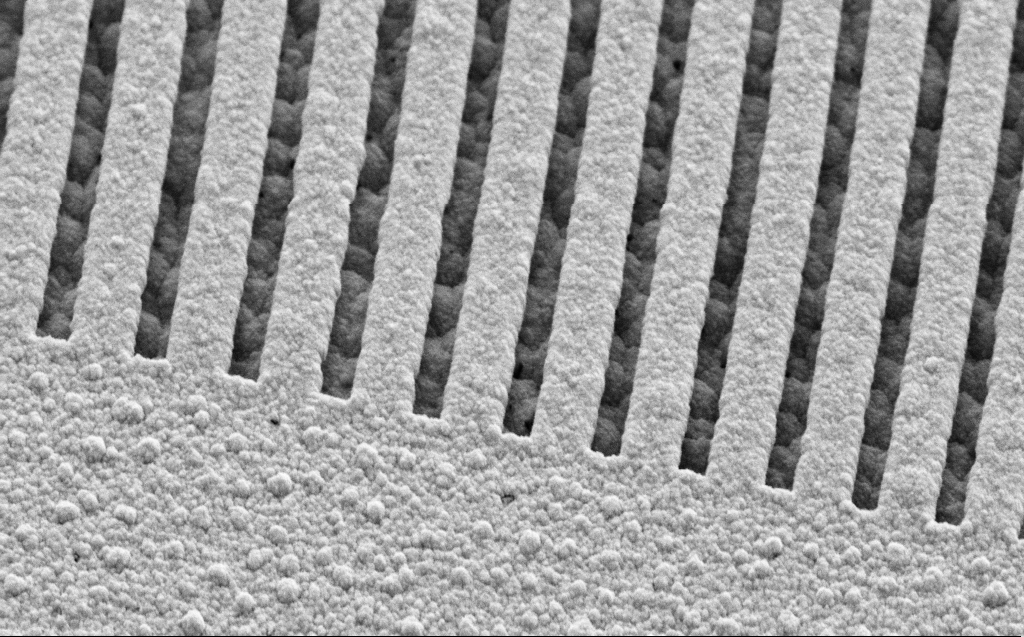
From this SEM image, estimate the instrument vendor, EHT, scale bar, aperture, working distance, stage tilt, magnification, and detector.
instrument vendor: Zeiss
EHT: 5 kV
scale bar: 1000 nm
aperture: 30 µm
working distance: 9 mm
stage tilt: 45°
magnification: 42.63 K X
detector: SE2